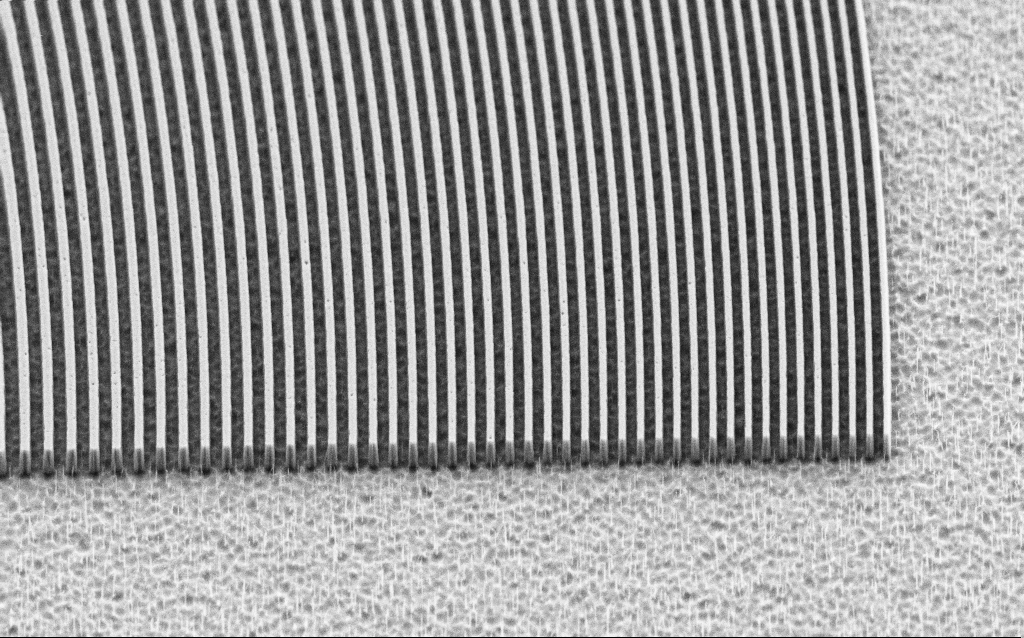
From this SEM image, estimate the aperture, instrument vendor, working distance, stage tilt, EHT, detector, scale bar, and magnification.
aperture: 30 µm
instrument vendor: Zeiss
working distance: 5 mm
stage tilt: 45°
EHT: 3 kV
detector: SE2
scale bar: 2000 nm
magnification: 15.82 K X